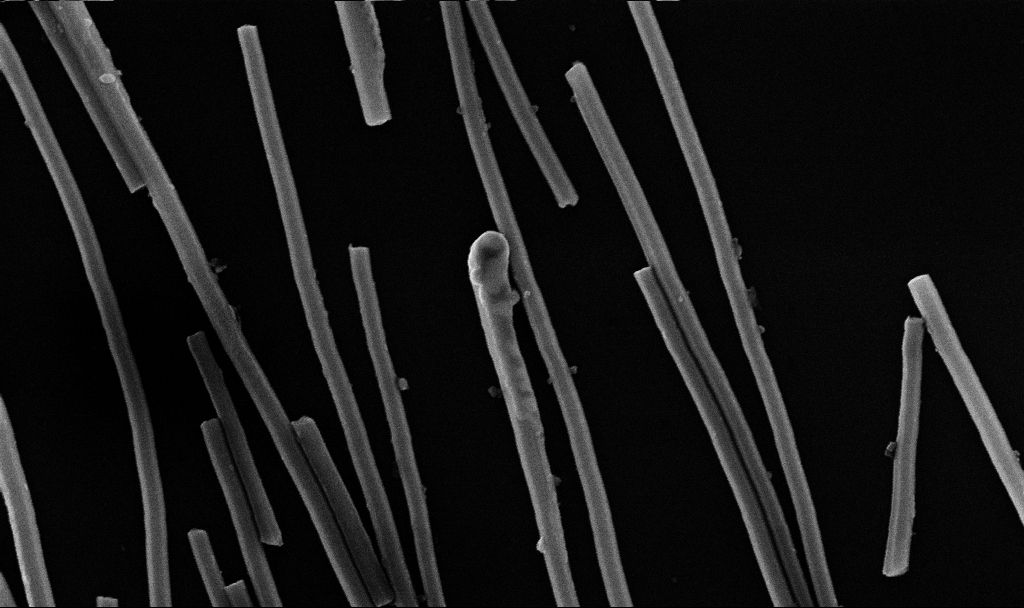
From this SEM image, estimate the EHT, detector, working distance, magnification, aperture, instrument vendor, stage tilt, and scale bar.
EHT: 10 kV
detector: InLens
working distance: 10.7 mm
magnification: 75.84 K X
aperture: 30 µm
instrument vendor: Zeiss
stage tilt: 0°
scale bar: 200 nm